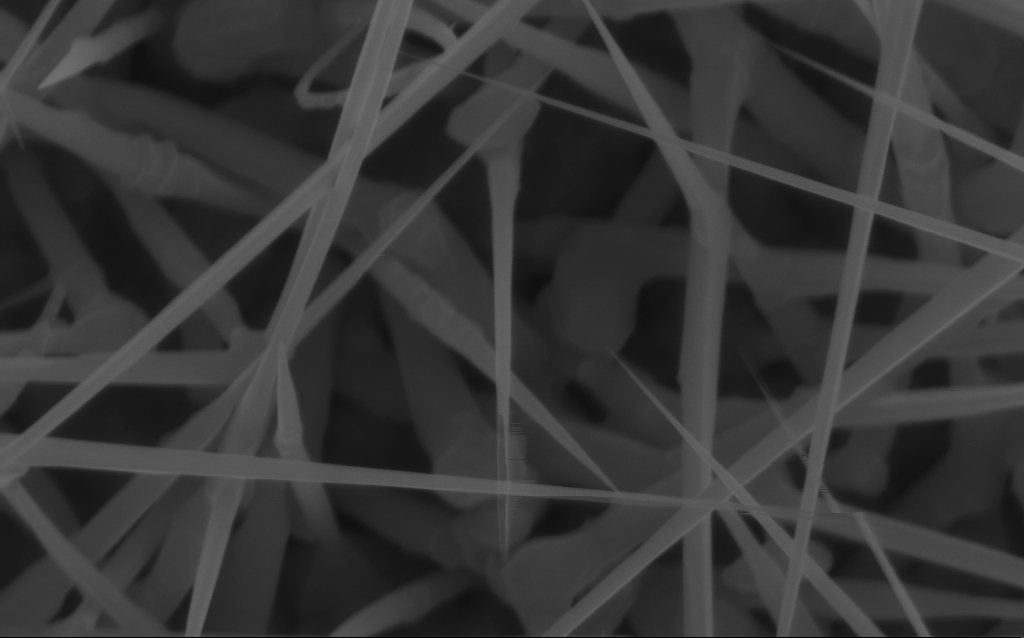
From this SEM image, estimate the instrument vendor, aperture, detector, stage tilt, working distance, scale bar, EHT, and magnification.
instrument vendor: Zeiss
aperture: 30 µm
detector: InLens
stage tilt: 0°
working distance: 7 mm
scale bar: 200 nm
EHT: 10 kV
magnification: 80 K X